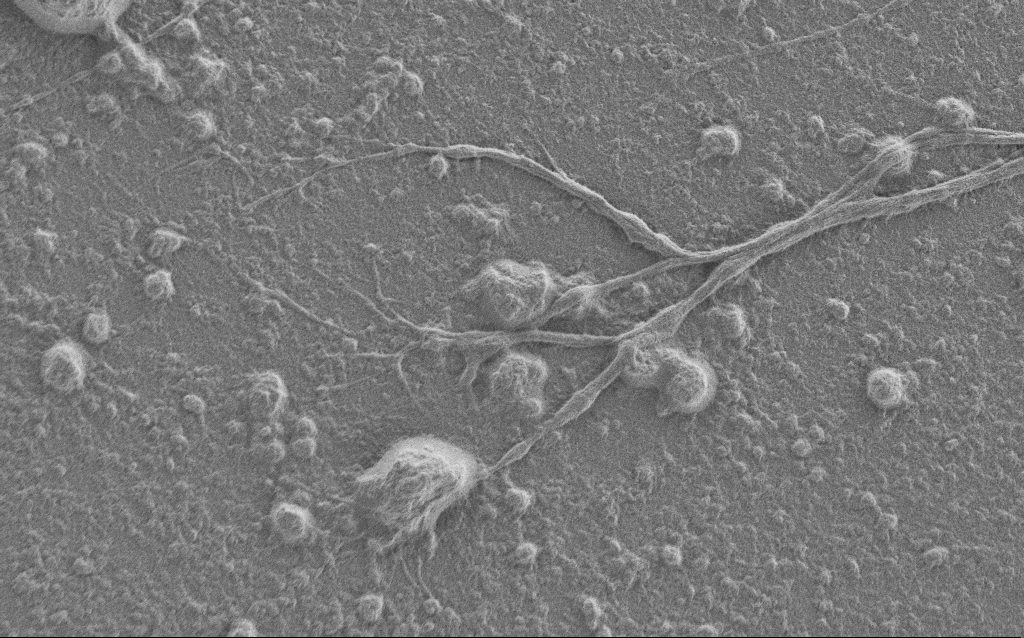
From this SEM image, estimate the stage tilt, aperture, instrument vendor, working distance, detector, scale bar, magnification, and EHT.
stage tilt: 0°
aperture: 30 µm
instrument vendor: Zeiss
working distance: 6 mm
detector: SE2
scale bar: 10000 nm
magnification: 5 K X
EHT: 1 kV